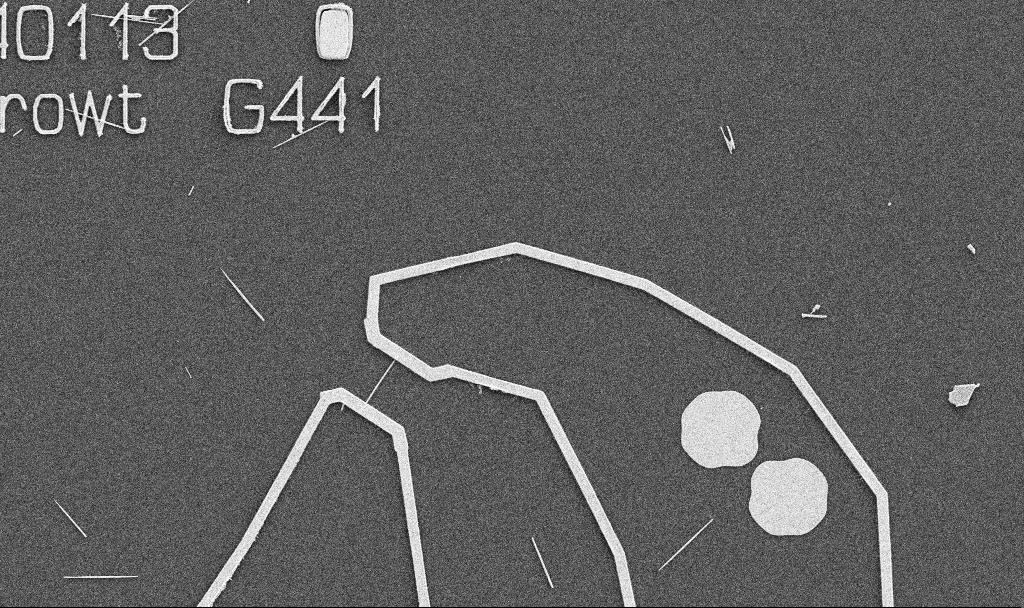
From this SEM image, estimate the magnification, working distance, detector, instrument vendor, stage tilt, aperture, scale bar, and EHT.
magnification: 5 K X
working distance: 10.7 mm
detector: SE2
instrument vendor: Zeiss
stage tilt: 0°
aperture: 30 µm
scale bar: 10000 nm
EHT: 5 kV